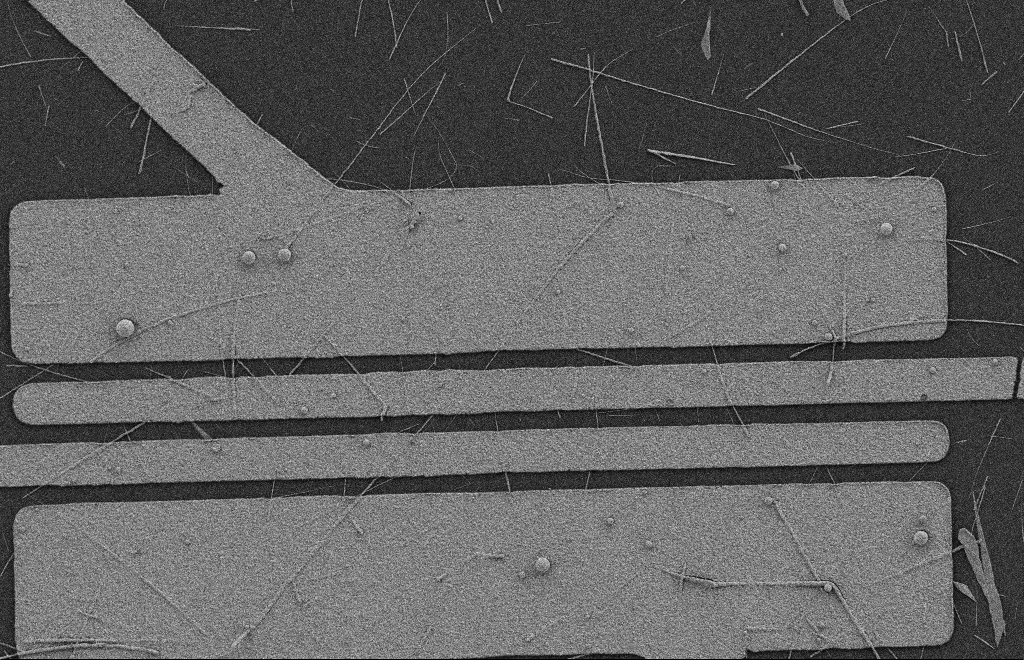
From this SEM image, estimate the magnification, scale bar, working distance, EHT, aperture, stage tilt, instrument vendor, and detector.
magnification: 5.6 K X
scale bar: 2000 nm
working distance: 8 mm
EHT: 2 kV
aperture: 20 µm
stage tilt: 0°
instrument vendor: Zeiss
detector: SE2